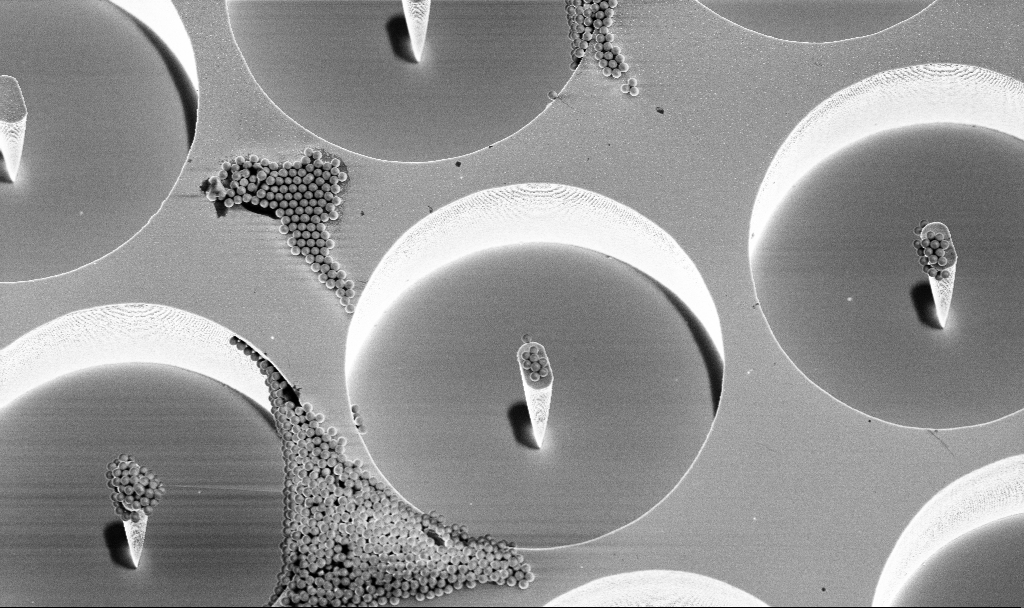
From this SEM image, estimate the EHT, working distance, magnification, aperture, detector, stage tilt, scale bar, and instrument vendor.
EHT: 4 kV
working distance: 4.7 mm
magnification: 4.69 K X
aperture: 30 µm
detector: InLens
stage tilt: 15°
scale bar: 10000 nm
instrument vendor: Zeiss